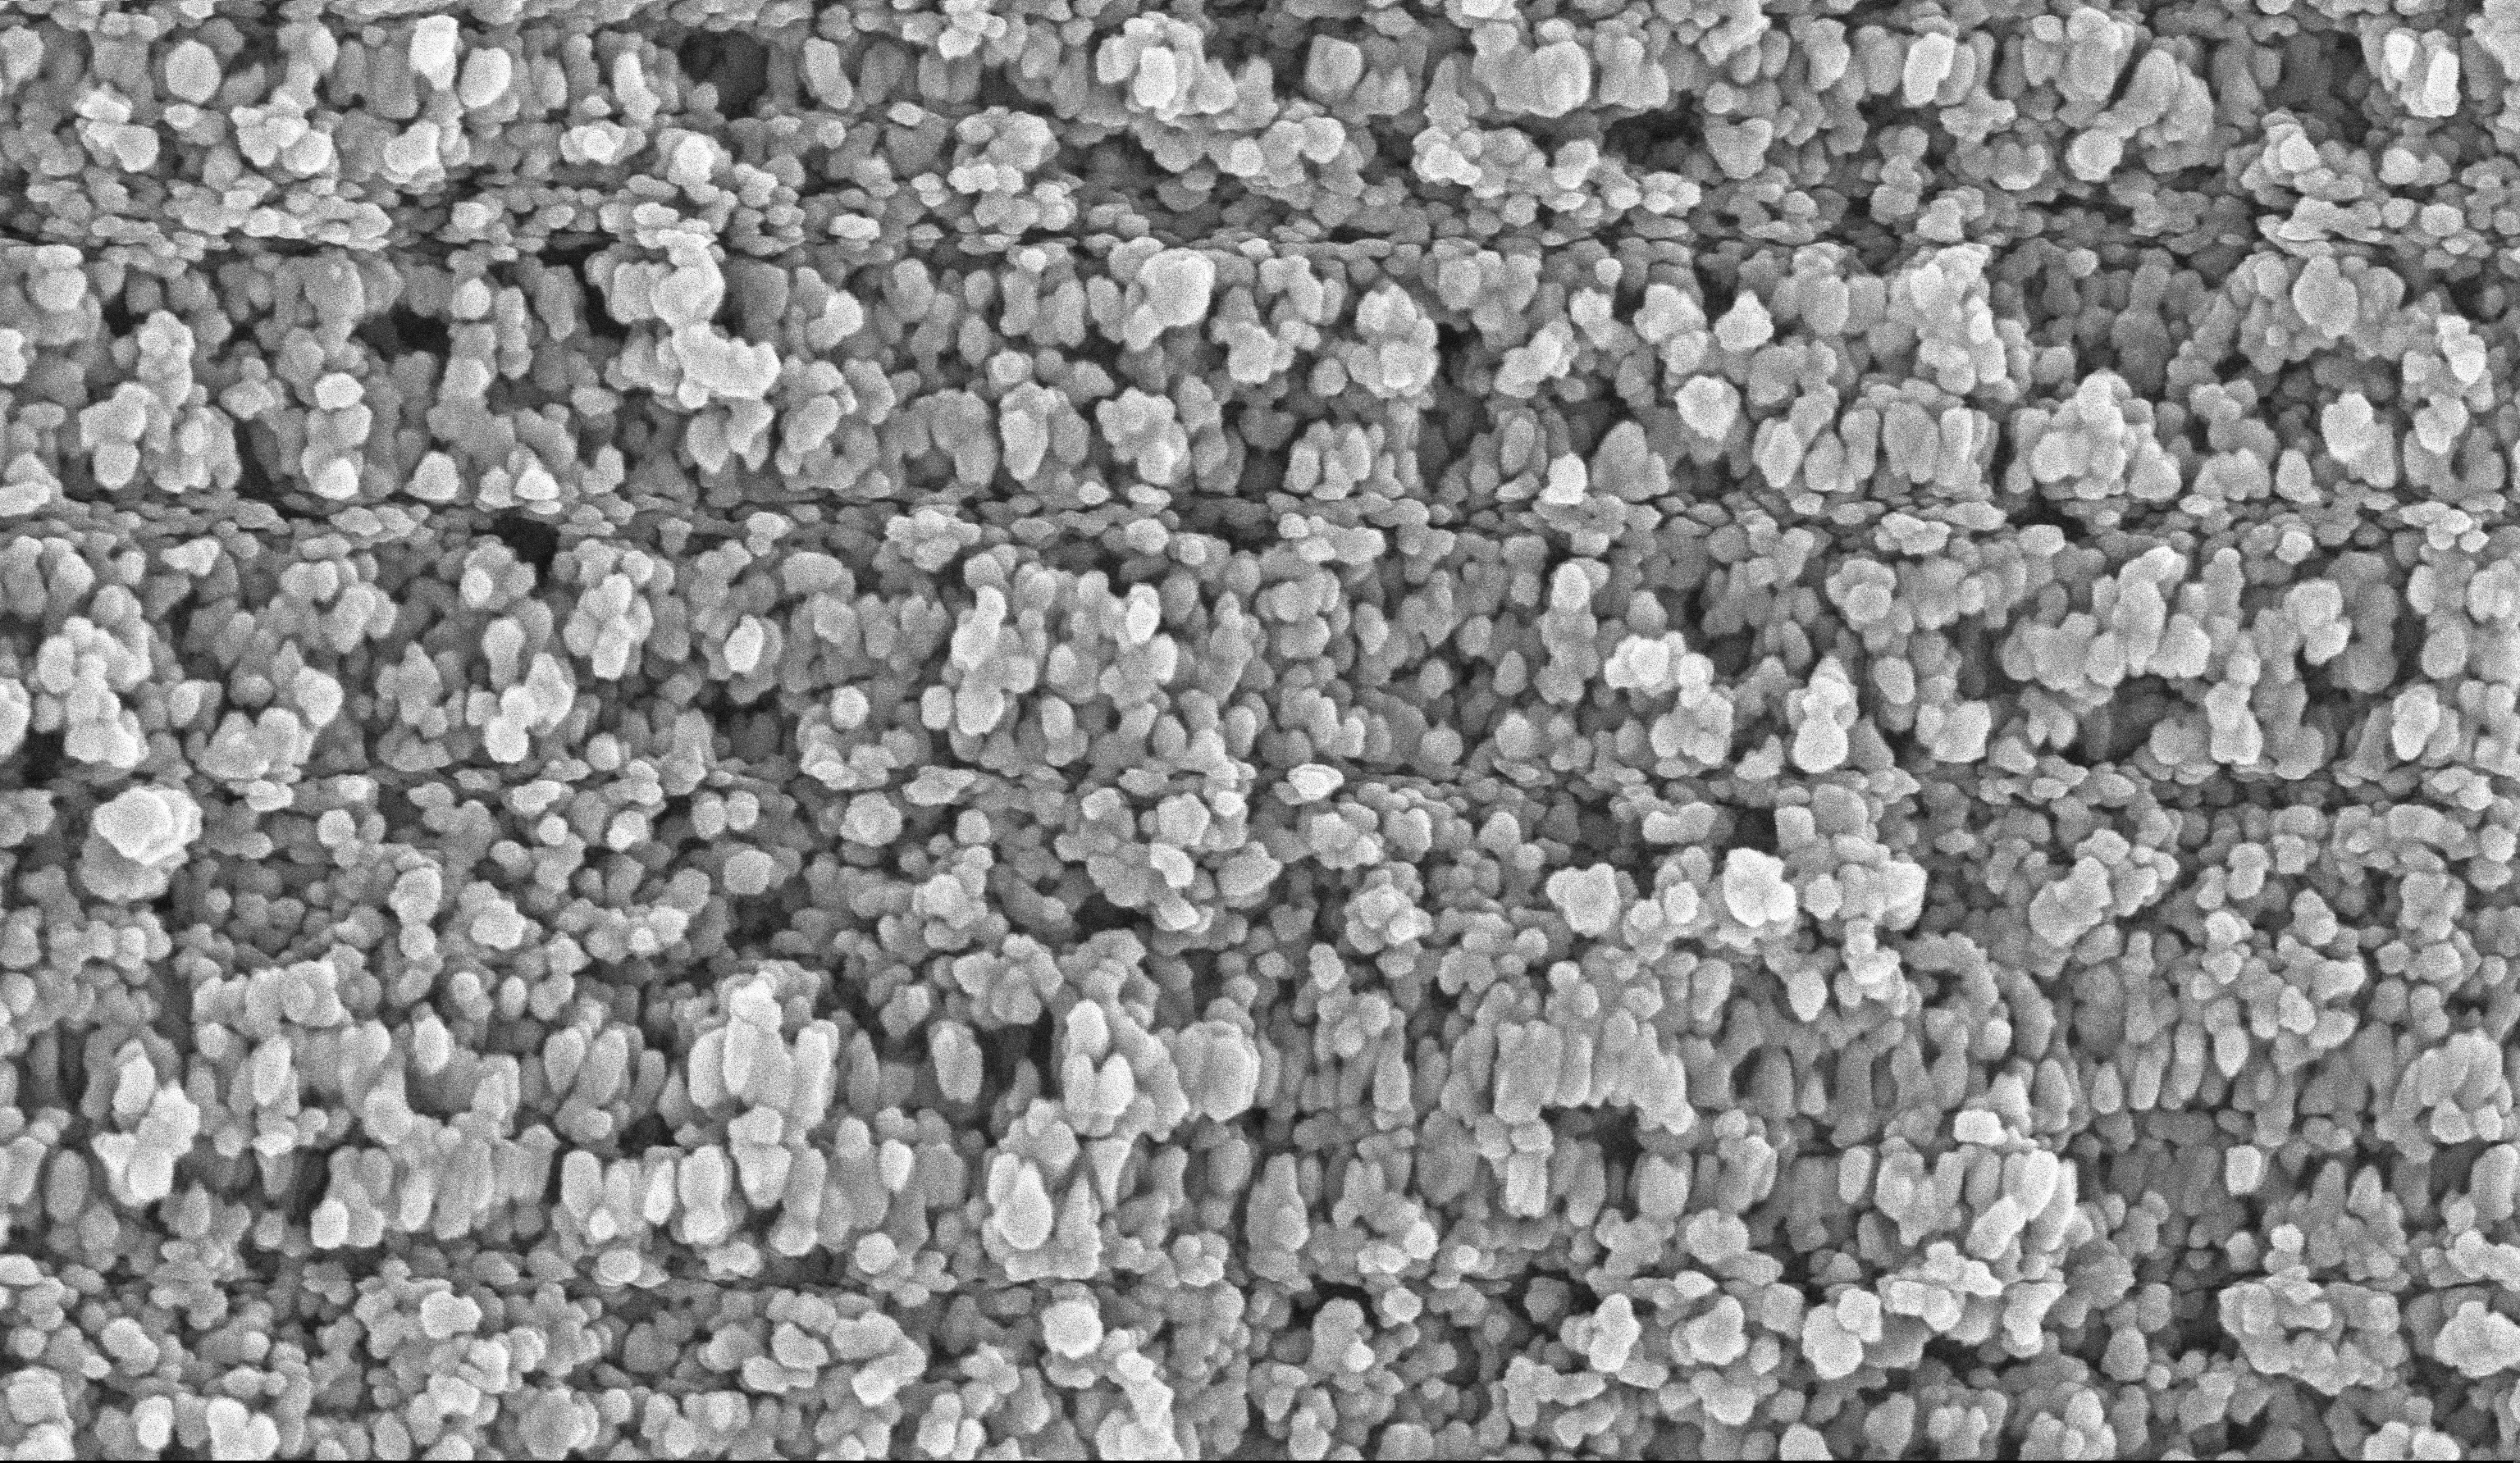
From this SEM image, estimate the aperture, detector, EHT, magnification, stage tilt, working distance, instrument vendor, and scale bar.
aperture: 30 µm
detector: InLens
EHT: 10 kV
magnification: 135 K X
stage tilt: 0°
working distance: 6 mm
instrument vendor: Zeiss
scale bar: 200 nm